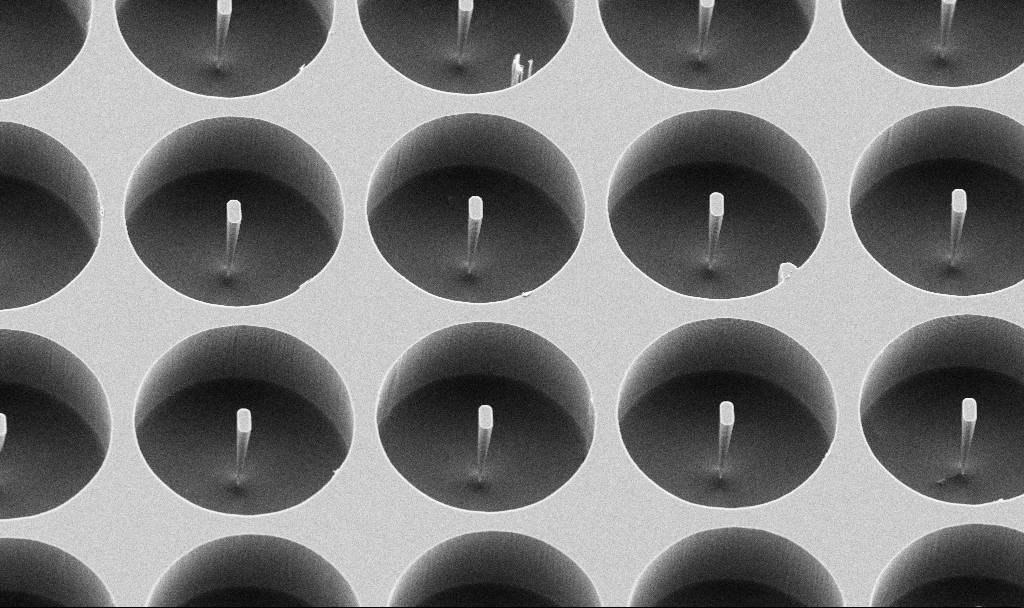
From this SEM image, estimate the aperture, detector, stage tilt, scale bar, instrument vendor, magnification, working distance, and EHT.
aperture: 30 µm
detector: SE2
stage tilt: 30°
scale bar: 10000 nm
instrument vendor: Zeiss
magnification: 2.71 K X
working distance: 7.4 mm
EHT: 5 kV